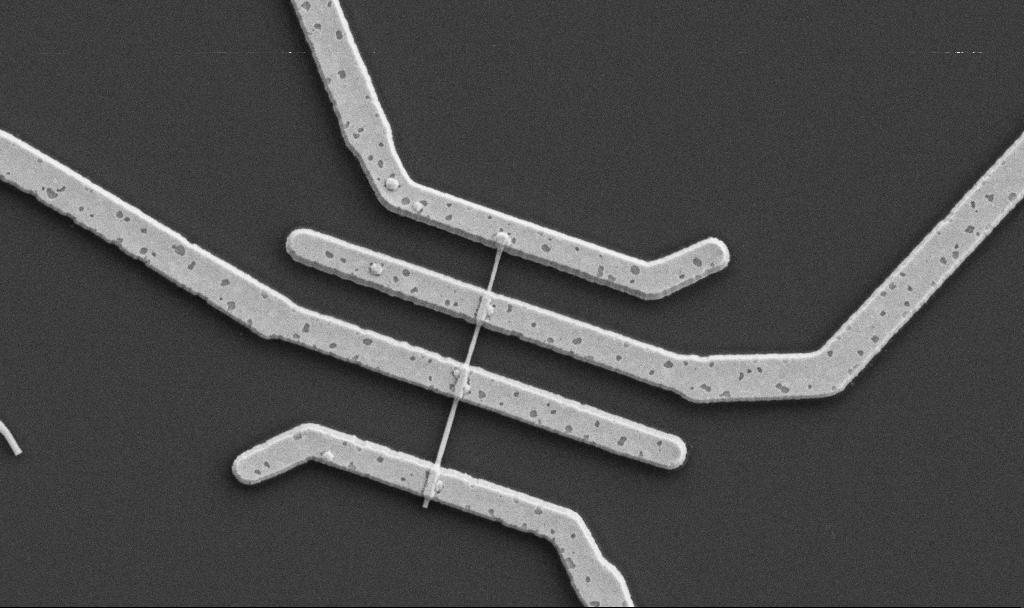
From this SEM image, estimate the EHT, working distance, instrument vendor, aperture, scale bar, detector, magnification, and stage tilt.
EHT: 5 kV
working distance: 10.7 mm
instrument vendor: Zeiss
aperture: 30 µm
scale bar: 1000 nm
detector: SE2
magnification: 20 K X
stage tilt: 0°